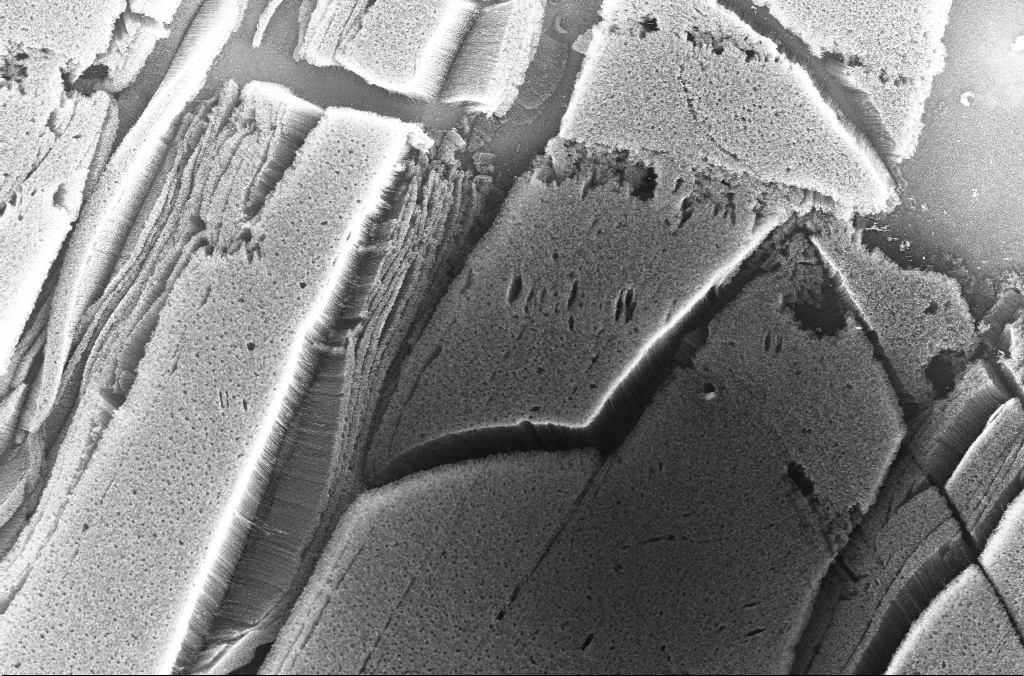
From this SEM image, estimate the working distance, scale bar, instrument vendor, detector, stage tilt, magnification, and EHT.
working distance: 4 mm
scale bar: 30000 nm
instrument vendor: Zeiss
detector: InLens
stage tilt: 0°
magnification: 1 K X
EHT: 10 kV